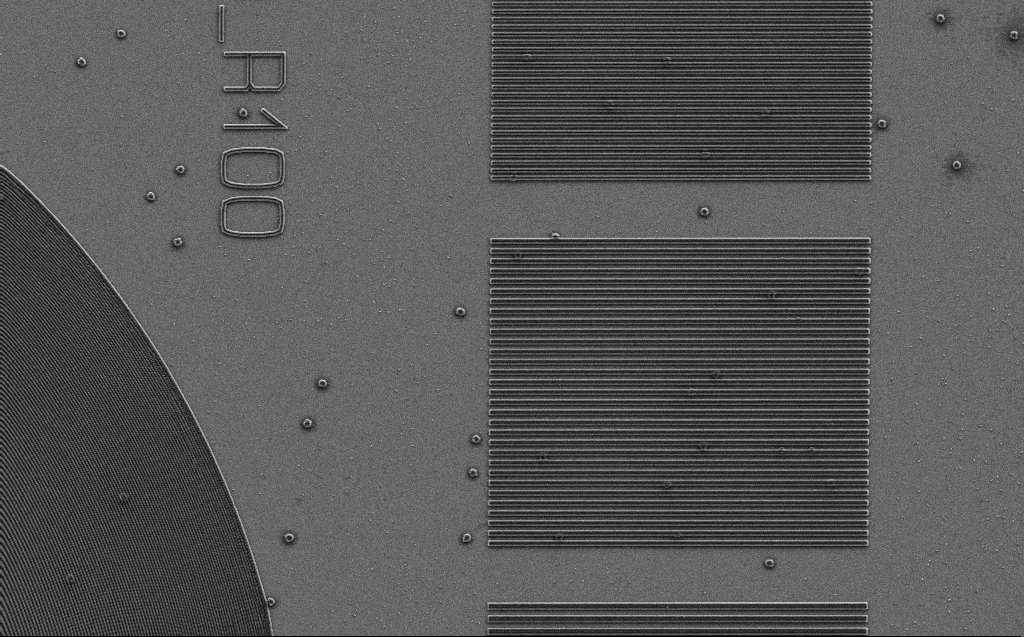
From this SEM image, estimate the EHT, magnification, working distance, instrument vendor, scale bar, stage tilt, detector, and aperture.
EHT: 3 kV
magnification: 4.69 K X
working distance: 6 mm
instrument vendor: Zeiss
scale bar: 10000 nm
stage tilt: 0°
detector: SE2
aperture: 30 µm